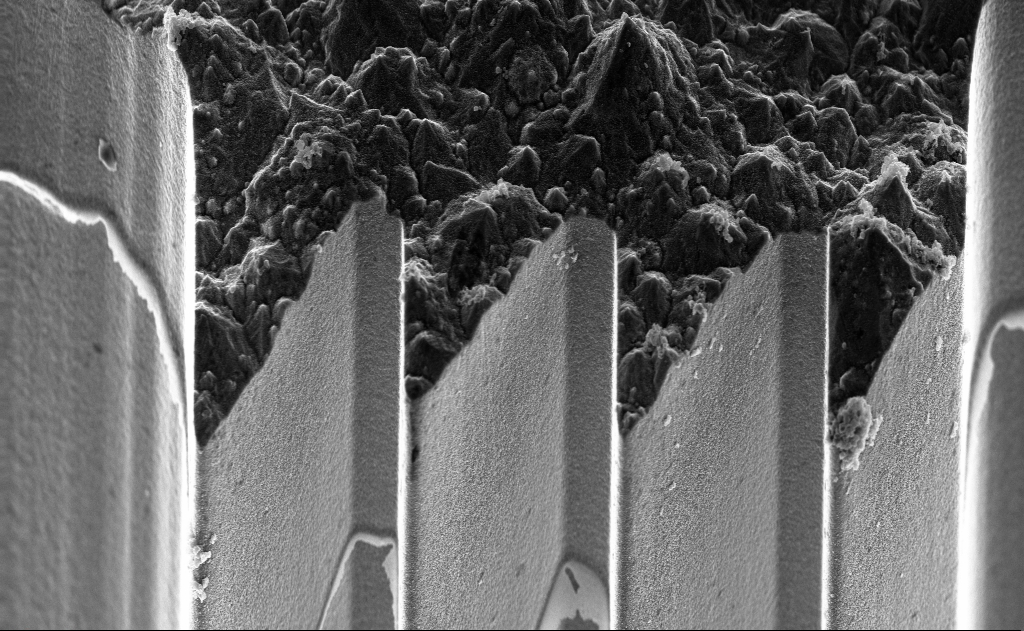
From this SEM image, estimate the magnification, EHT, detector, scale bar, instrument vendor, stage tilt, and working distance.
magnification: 6.8 K X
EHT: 10 kV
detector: InLens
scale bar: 10000 nm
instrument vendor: Zeiss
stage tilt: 45°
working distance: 4 mm